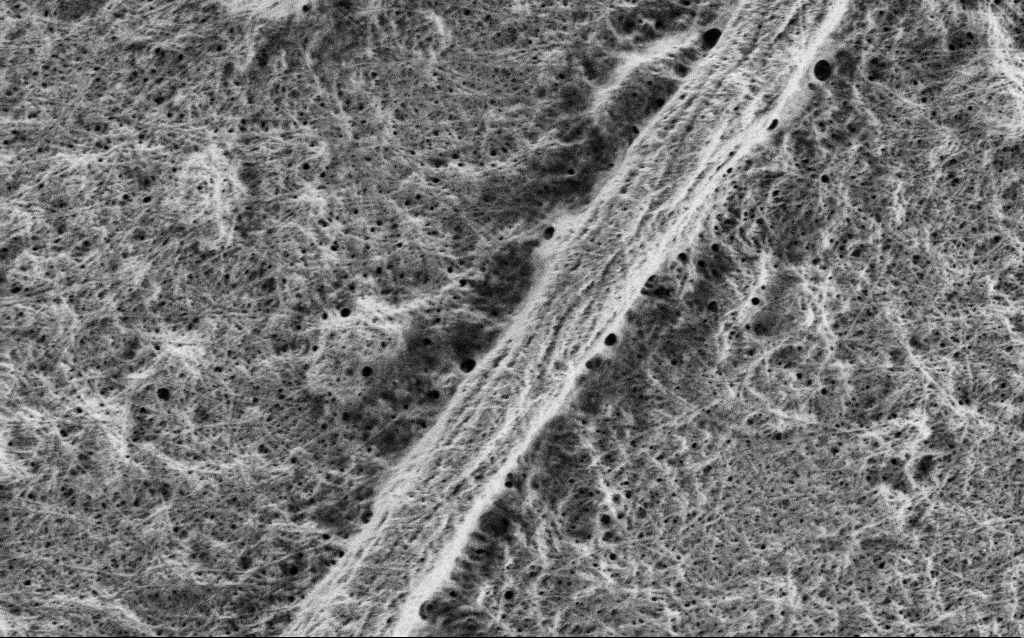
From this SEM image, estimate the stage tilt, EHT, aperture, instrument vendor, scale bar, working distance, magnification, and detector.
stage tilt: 0°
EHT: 1 kV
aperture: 30 µm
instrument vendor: Zeiss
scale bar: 2000 nm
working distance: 4 mm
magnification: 20 K X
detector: SE2